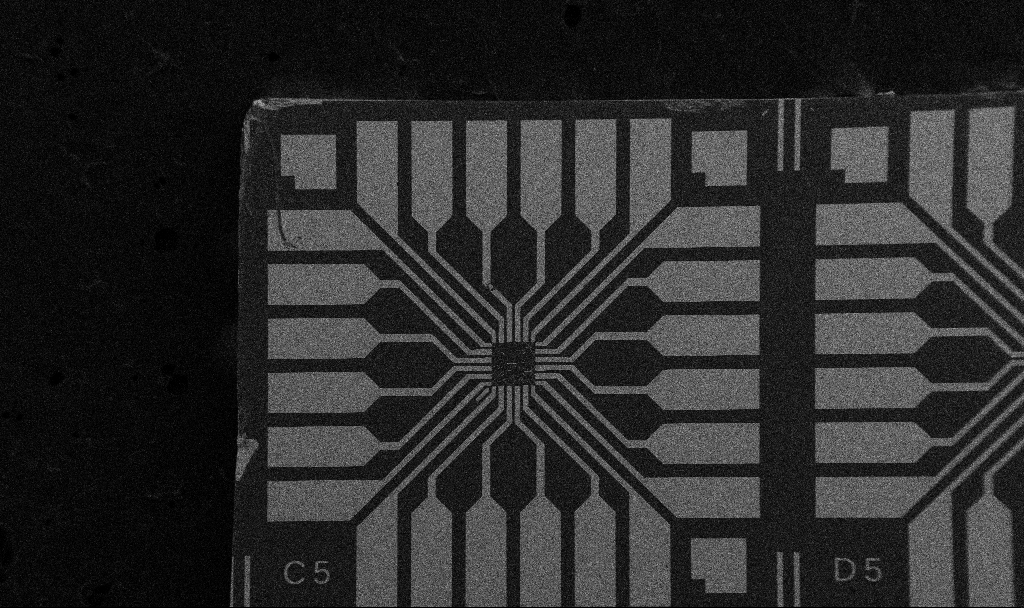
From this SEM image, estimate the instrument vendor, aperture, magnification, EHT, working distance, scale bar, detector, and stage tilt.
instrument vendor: Zeiss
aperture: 30 µm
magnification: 0.1 K X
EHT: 5 kV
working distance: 10.7 mm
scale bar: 200000 nm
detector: SE2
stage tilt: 0°